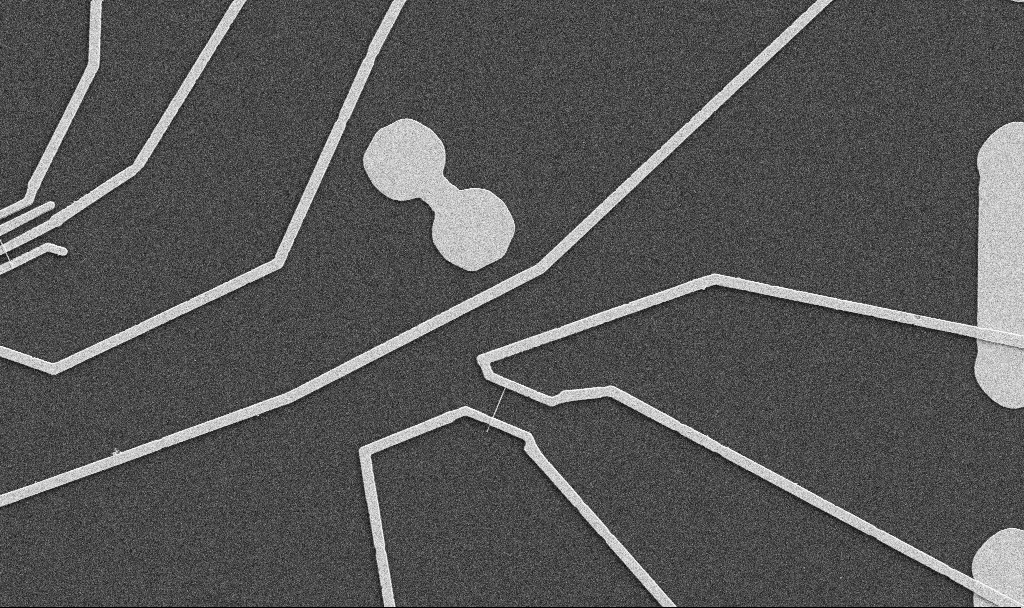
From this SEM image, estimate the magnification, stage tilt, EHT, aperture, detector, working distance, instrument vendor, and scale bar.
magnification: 5 K X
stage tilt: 0°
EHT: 5 kV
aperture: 30 µm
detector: SE2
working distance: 10.7 mm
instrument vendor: Zeiss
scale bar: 10000 nm